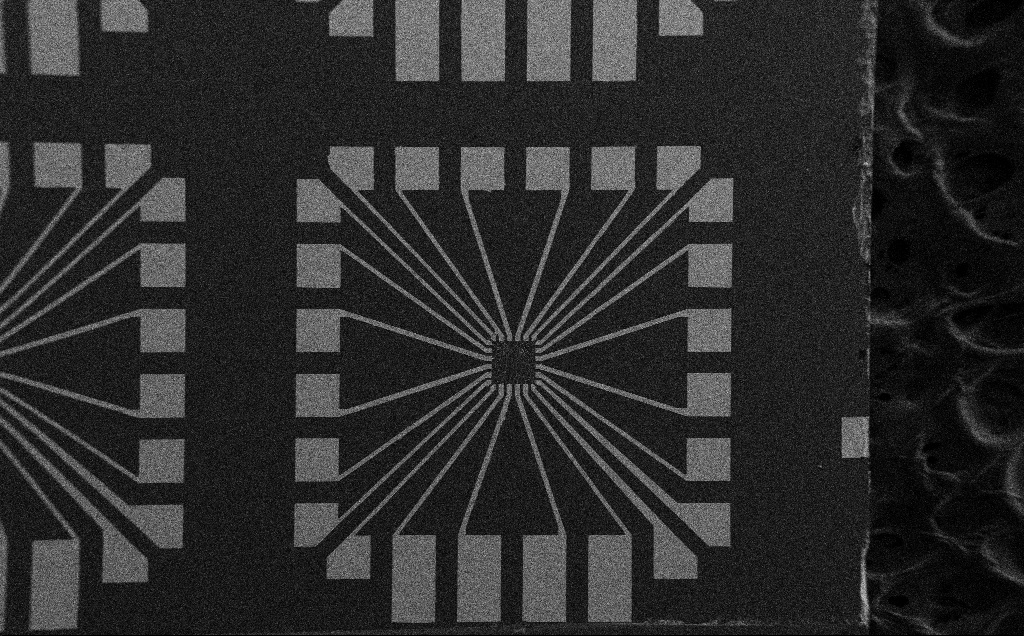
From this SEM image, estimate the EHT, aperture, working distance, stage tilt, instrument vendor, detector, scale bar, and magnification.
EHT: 5 kV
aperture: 30 µm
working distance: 10 mm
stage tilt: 0°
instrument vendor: Zeiss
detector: SE2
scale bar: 200000 nm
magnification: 0.1 K X